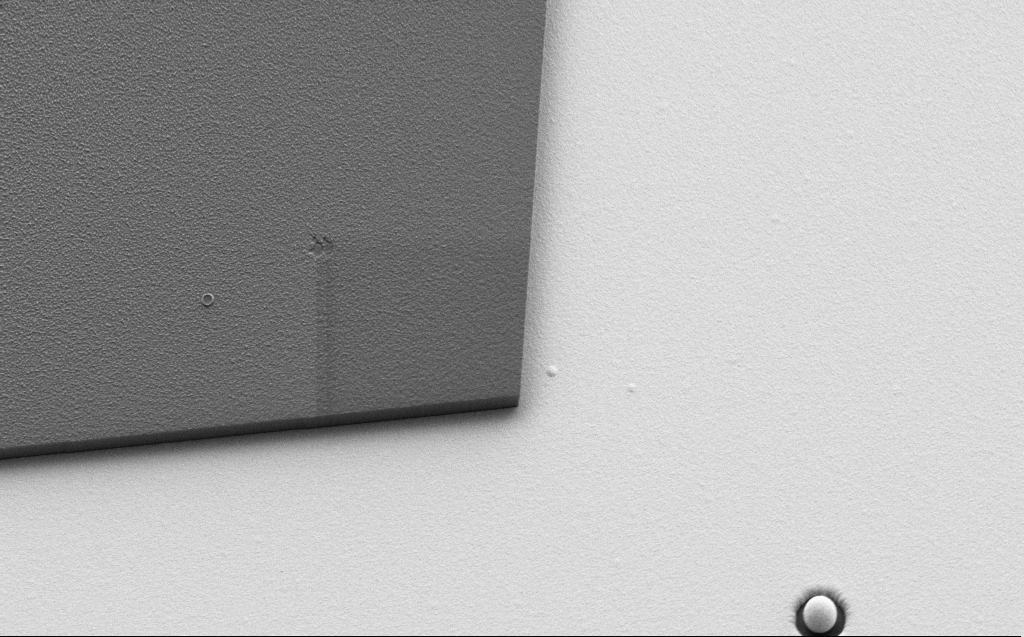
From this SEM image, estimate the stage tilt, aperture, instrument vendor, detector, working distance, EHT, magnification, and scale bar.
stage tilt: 30°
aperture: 30 µm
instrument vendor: Zeiss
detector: SE2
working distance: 6 mm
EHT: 1.1 kV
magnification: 5.22 K X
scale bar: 10000 nm